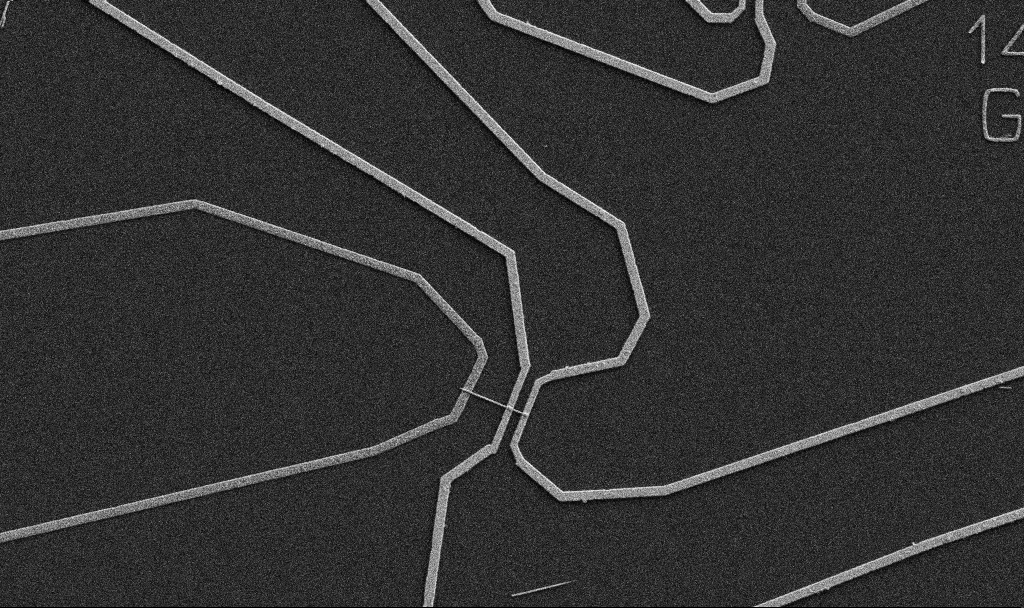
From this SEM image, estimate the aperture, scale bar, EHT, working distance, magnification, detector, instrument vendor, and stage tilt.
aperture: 30 µm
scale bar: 10000 nm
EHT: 5 kV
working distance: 10.7 mm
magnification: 5 K X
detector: SE2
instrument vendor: Zeiss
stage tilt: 0°